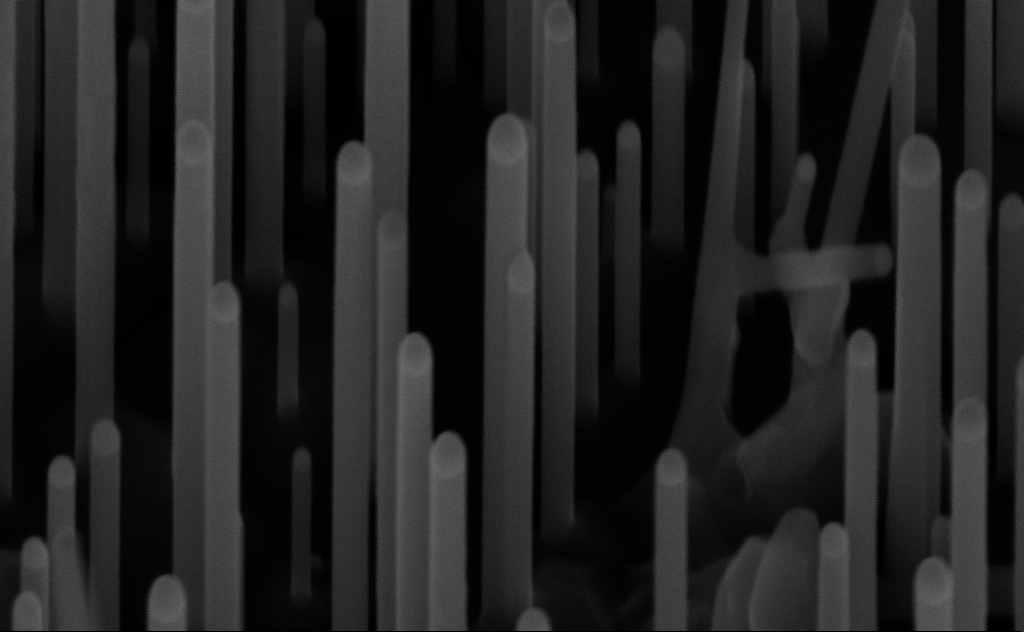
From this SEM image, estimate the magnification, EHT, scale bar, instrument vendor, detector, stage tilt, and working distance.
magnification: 213.61 K X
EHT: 10 kV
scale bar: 200 nm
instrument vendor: Zeiss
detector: InLens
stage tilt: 45°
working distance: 7 mm